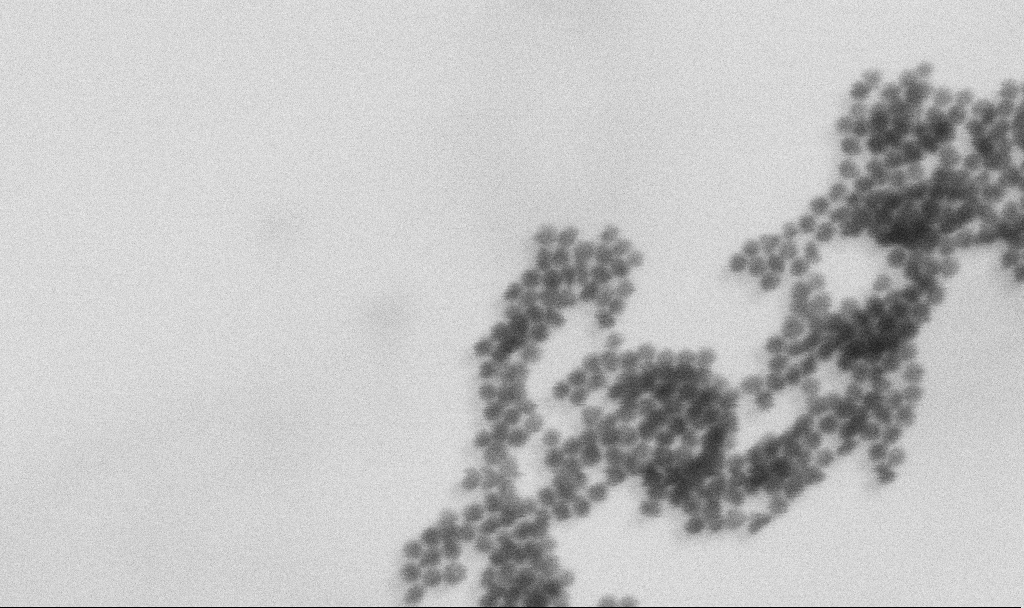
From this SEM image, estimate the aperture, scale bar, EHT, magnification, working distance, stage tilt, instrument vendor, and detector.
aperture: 30 µm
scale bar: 100 nm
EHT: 6 kV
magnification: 400 K X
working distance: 7.2 mm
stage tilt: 0°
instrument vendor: Zeiss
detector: SE2